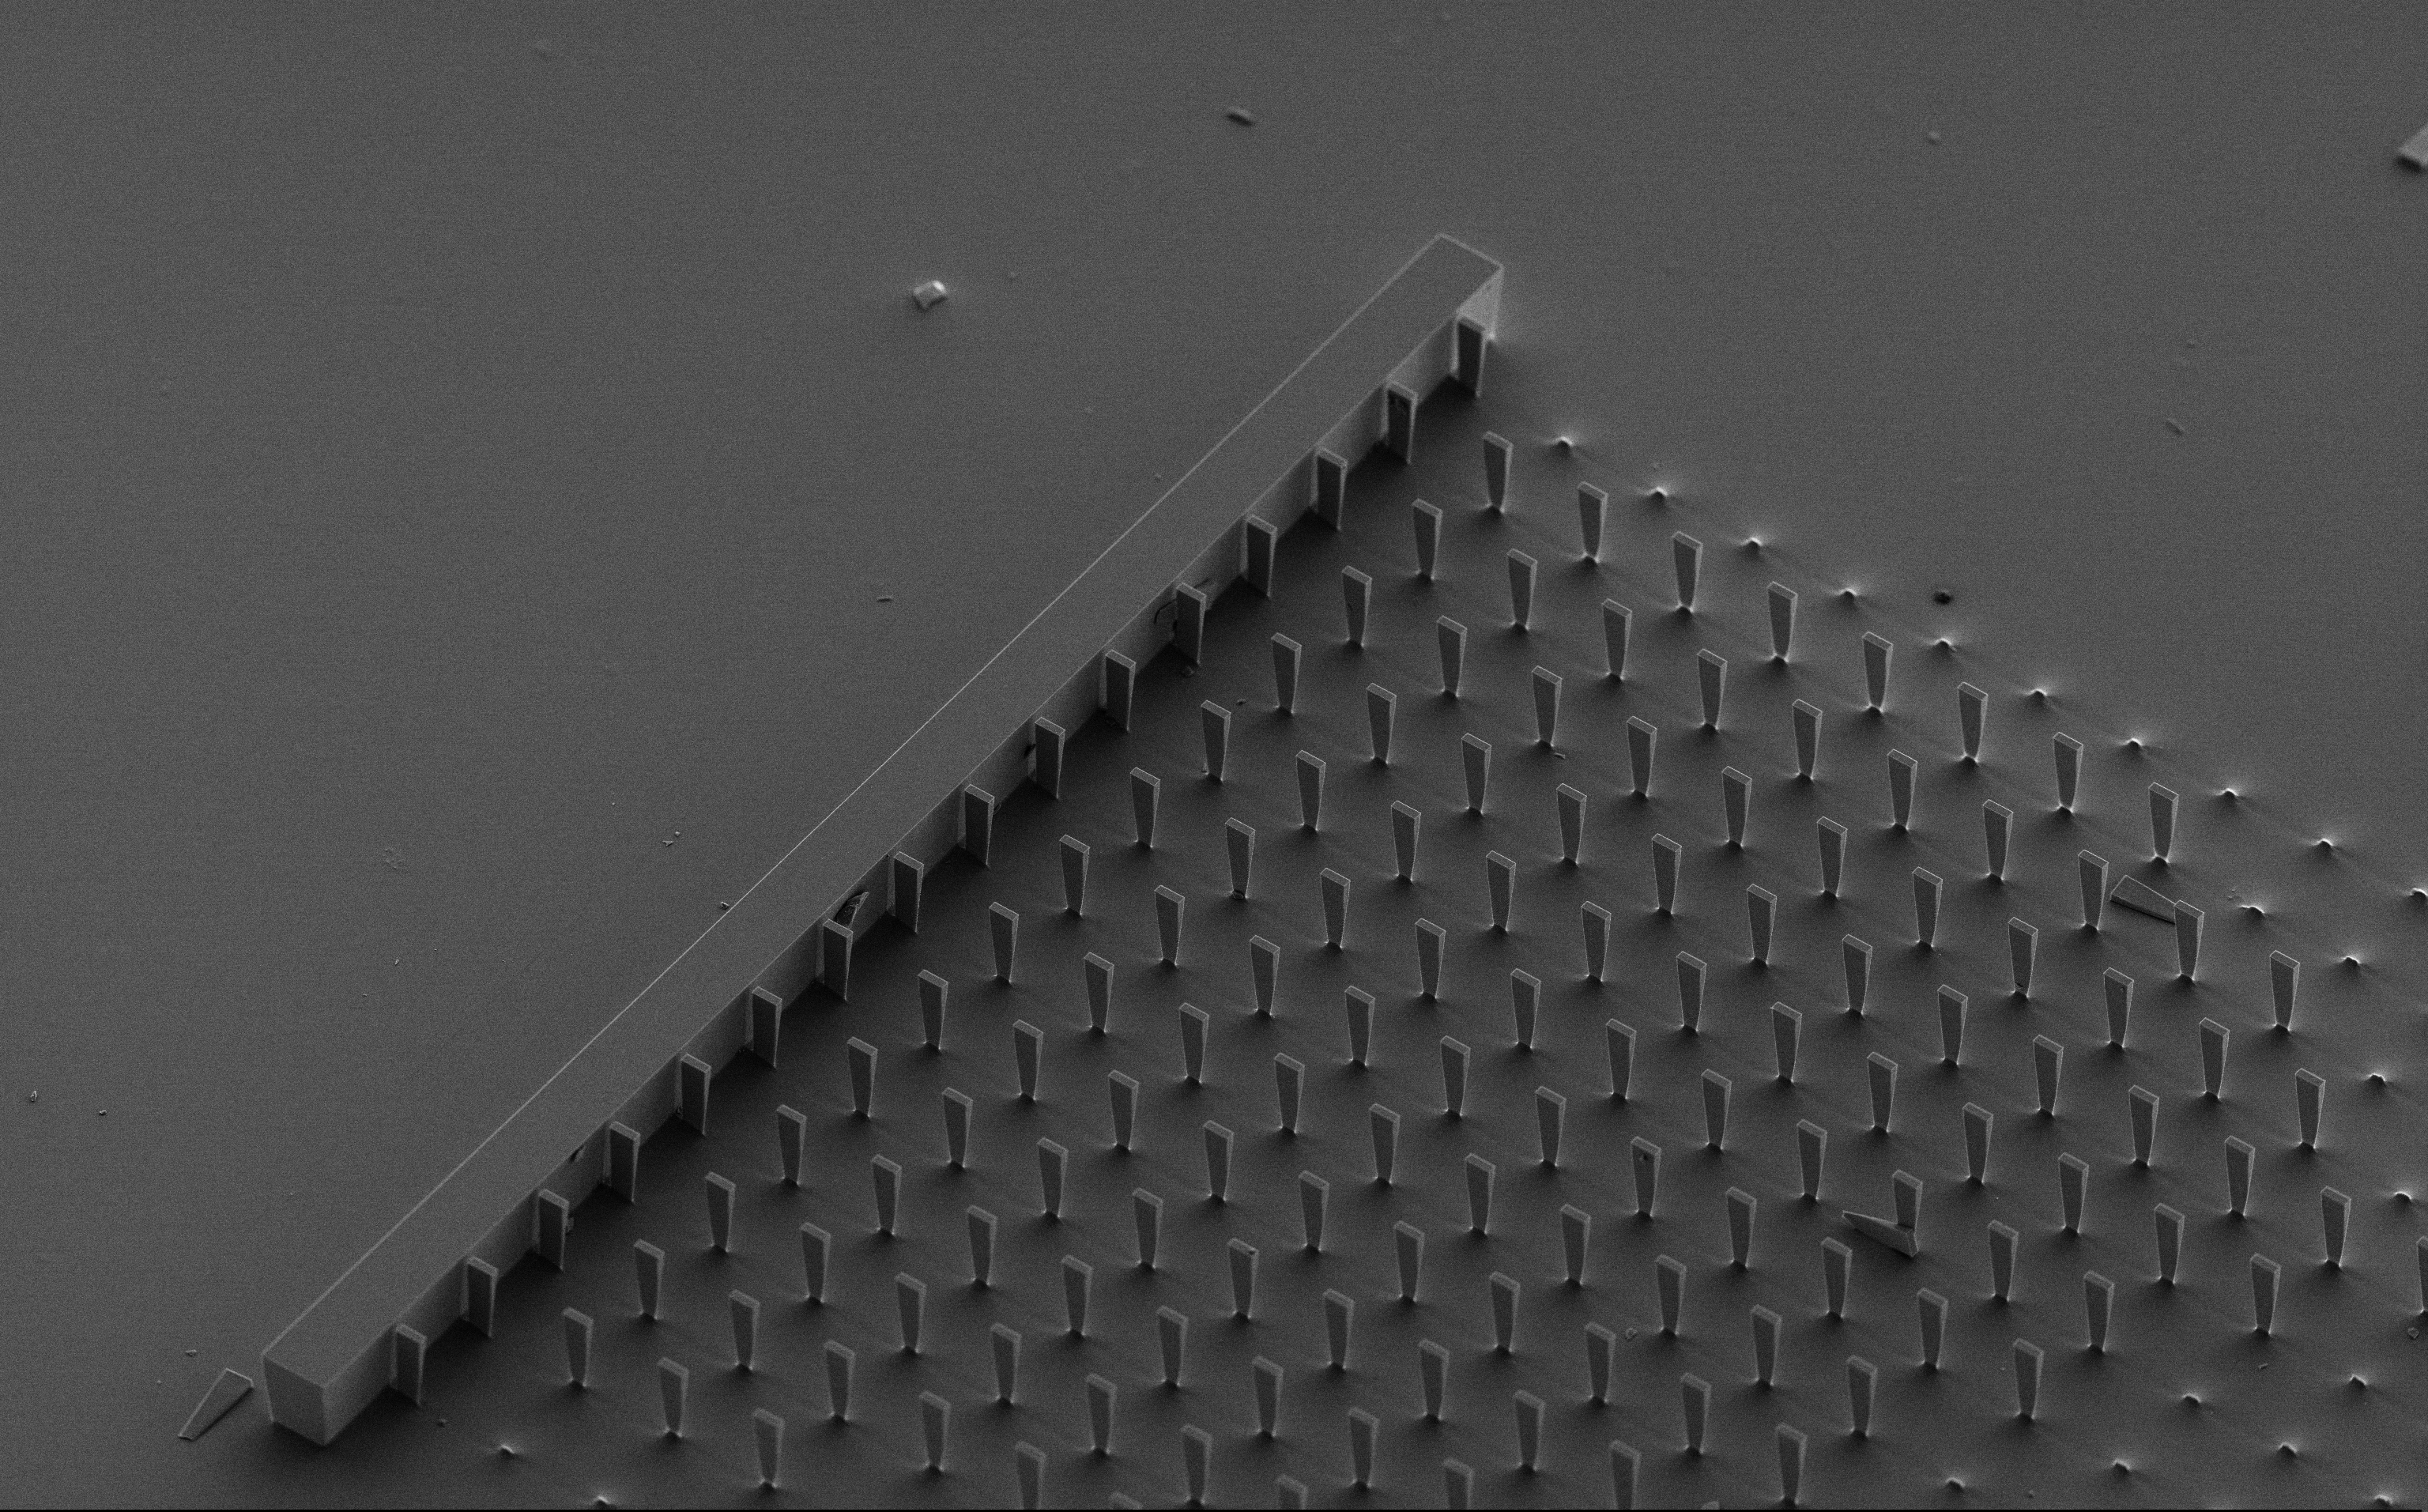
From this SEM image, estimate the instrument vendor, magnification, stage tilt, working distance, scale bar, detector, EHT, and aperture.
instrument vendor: Zeiss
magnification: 0.614 K X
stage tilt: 45°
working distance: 10 mm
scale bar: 100000 nm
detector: SE2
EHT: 5 kV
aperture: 30 µm